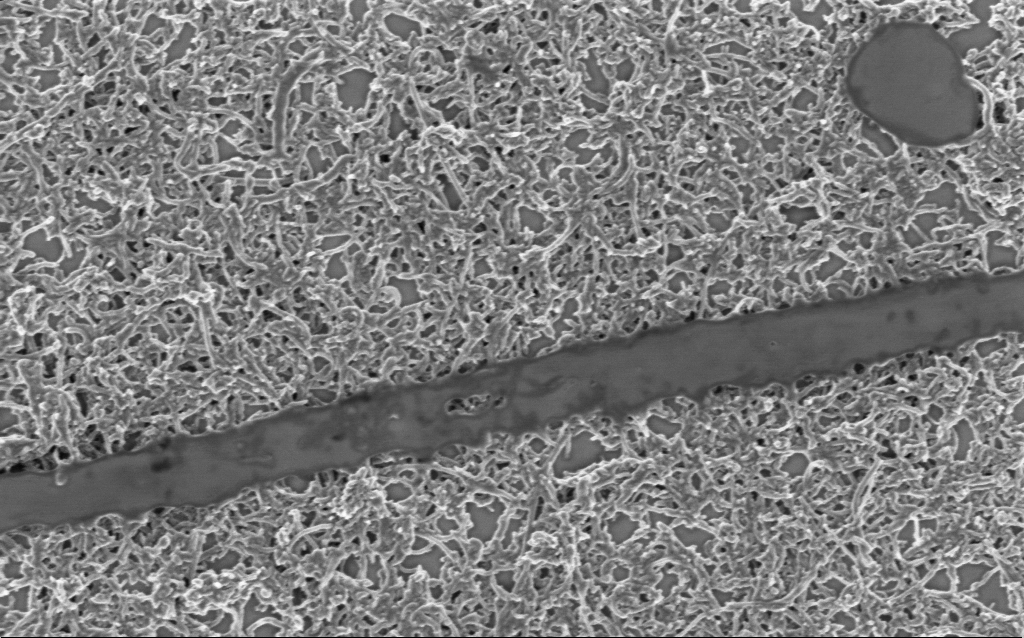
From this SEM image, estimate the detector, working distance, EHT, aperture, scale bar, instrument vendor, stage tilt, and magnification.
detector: InLens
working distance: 7 mm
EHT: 1 kV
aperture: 30 µm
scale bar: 1000 nm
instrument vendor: Zeiss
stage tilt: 0°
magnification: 50 K X